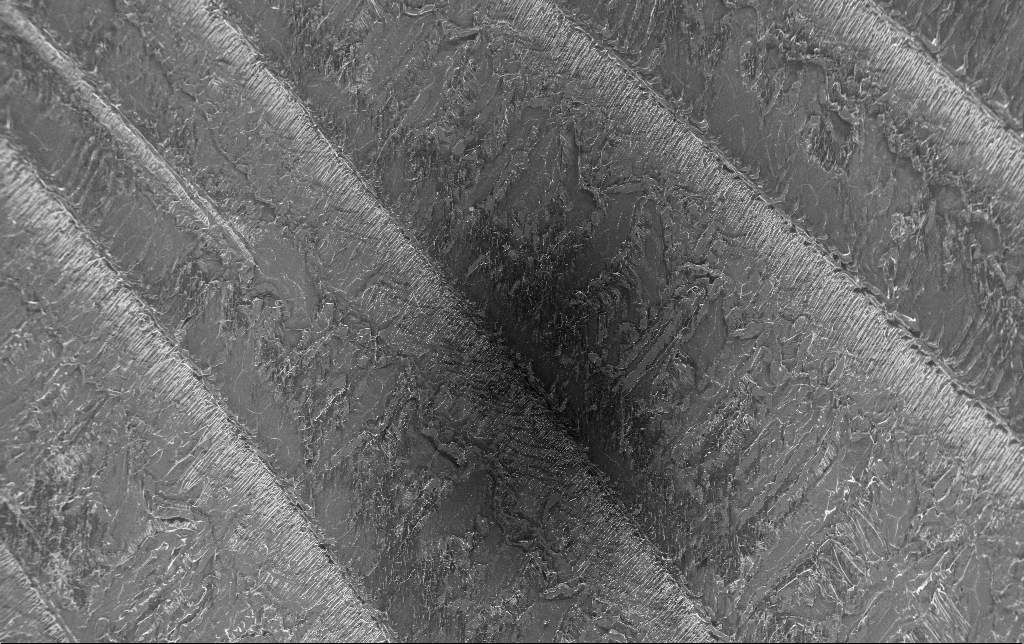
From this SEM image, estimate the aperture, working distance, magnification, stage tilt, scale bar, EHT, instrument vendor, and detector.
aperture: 30 µm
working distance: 3.1 mm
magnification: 0.176 K X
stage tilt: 0°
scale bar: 100000 nm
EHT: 3 kV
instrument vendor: Zeiss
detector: InLens